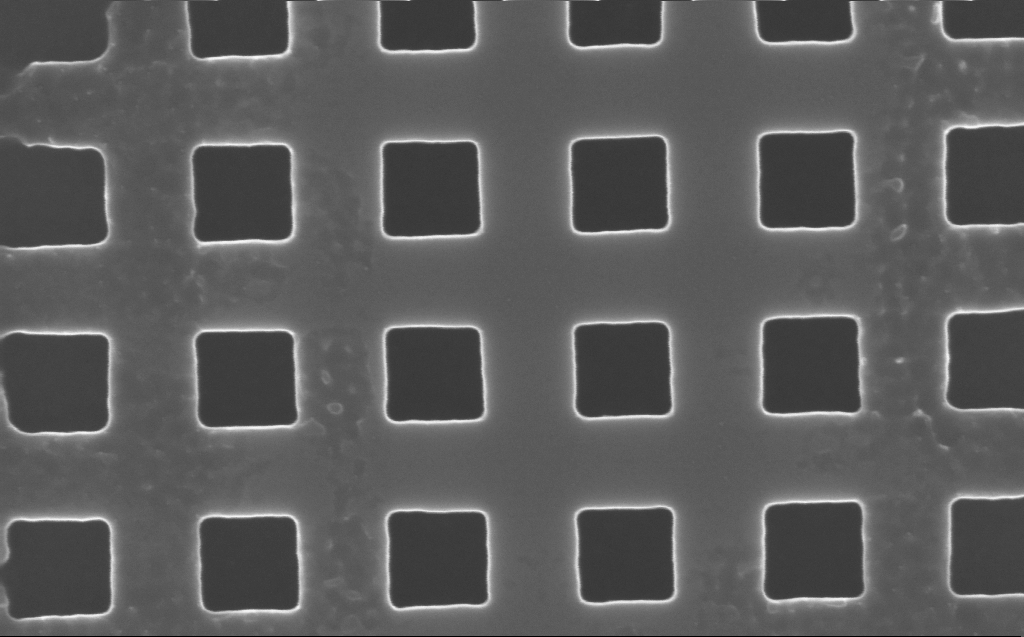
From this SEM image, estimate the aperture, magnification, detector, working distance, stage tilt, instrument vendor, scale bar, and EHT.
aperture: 30 µm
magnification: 140 K X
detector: InLens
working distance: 4 mm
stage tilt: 0°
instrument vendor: Zeiss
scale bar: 100 nm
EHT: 10 kV